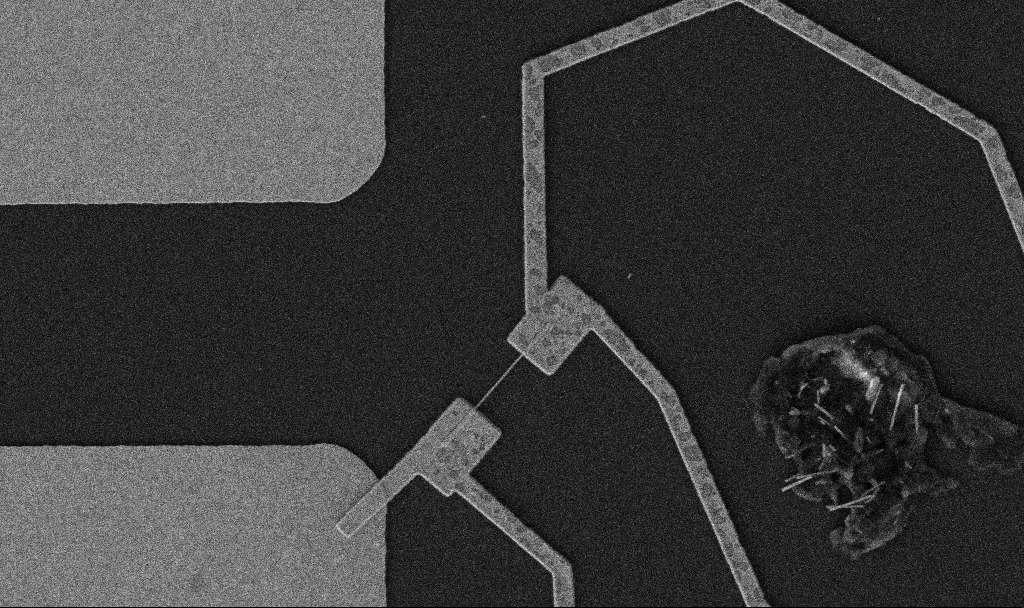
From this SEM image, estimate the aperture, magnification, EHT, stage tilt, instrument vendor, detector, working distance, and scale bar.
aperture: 30 µm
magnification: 10 K X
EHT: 5 kV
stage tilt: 0°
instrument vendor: Zeiss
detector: SE2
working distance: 10.7 mm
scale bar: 2000 nm